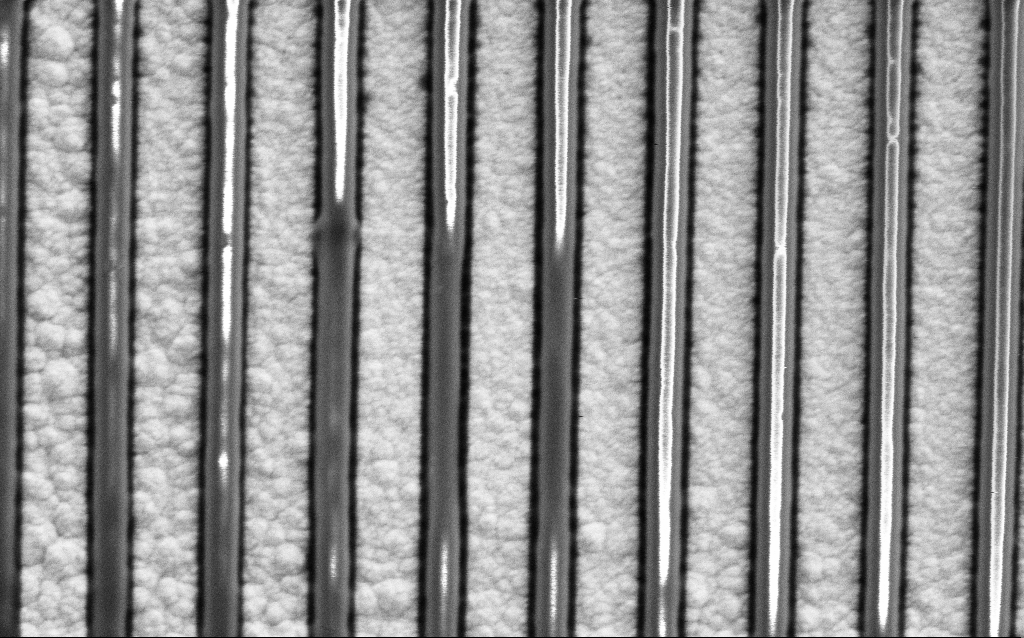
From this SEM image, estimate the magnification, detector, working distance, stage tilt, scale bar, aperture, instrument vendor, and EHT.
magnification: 65.81 K X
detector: SE2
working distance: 7.9 mm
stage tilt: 0°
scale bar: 1000 nm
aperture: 30 µm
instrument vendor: Zeiss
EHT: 1.5 kV